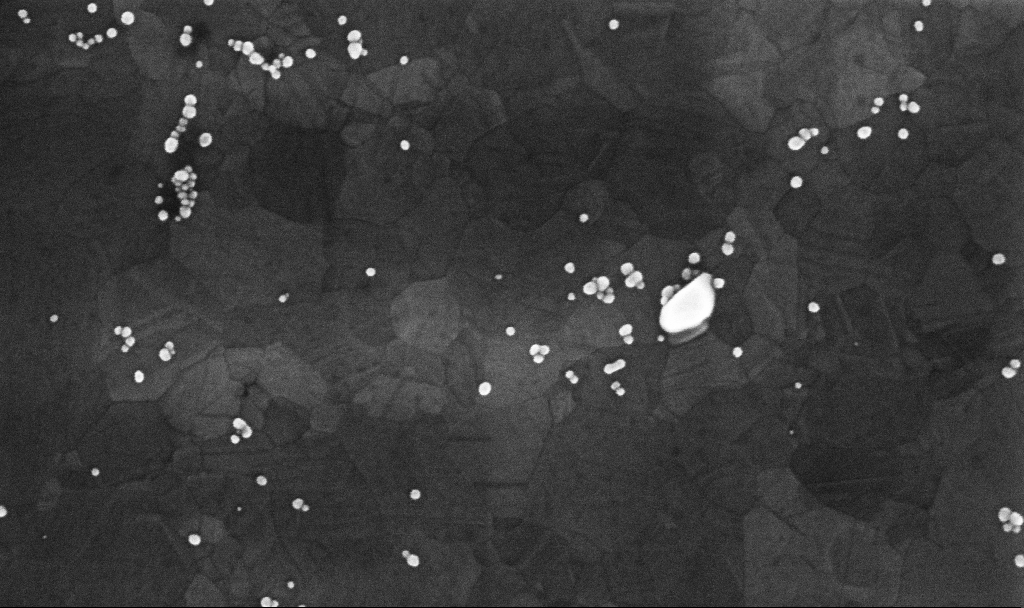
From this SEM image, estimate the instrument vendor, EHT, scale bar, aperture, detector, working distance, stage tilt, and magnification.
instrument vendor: Zeiss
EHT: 10 kV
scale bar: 200 nm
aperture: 30 µm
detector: InLens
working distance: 3.4 mm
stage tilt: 0°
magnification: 179.42 K X